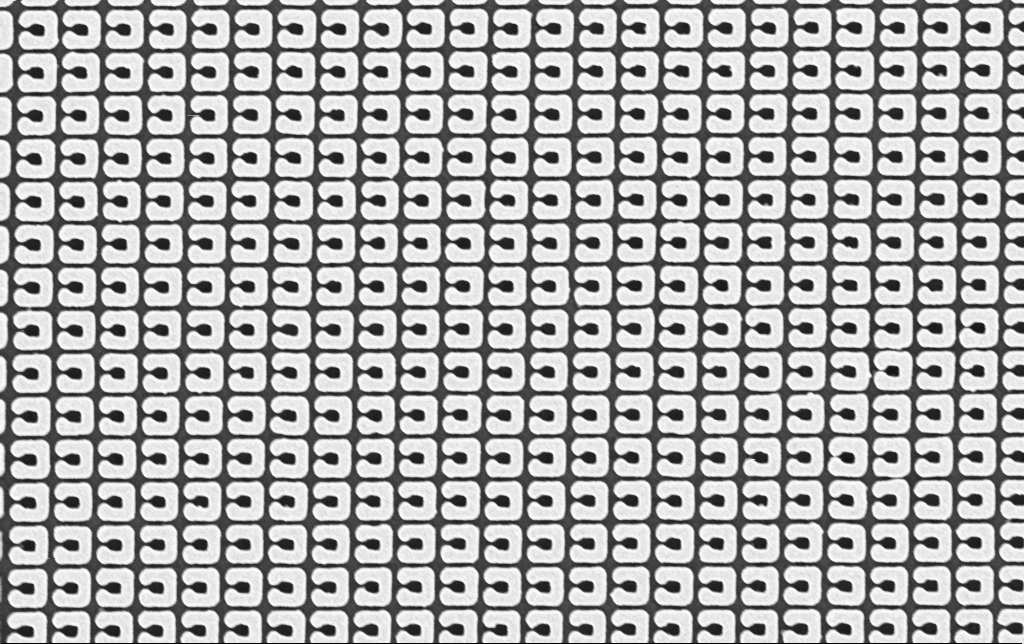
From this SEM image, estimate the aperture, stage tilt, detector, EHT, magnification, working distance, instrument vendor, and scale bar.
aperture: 30 µm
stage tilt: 0°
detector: InLens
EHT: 3 kV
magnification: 34.52 K X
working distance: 3.5 mm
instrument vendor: Zeiss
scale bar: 2000 nm